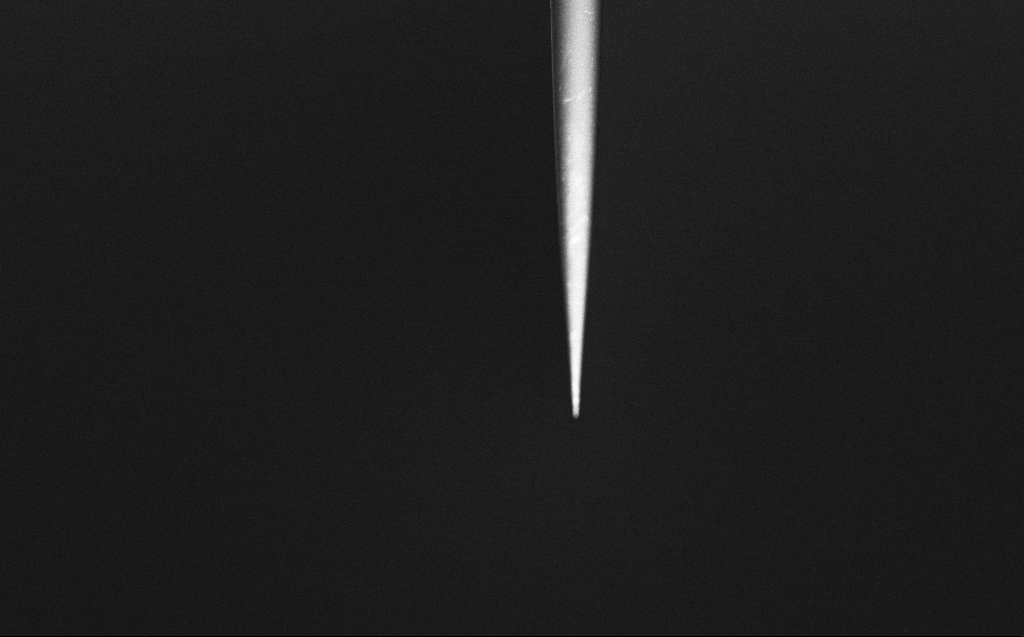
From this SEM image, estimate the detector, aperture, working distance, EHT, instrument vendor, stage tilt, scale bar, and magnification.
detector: InLens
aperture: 30 µm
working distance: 4 mm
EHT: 2 kV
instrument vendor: Zeiss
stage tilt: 45°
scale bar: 20000 nm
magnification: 1 K X